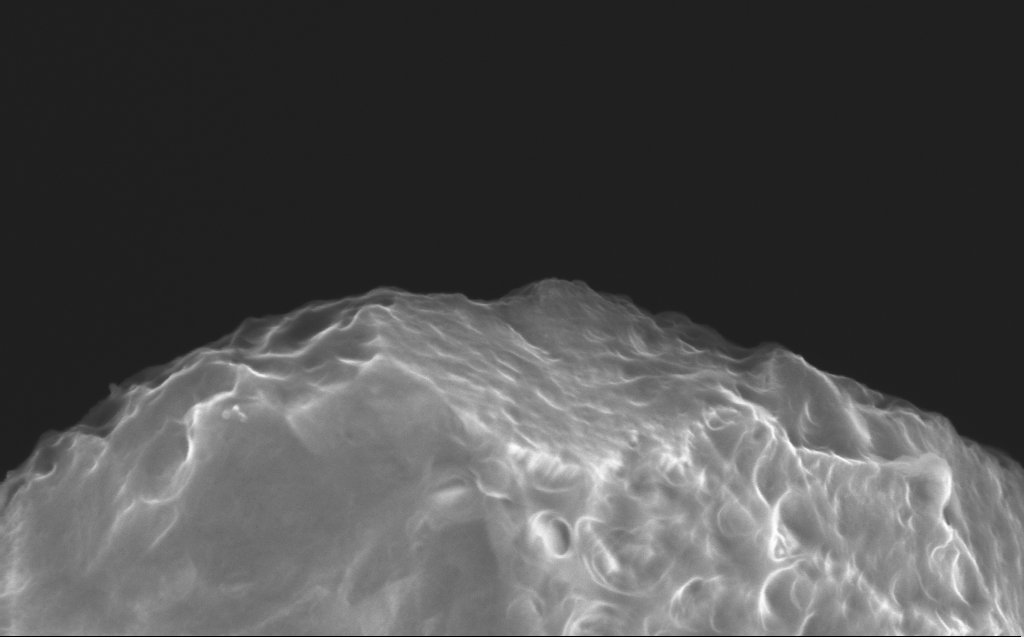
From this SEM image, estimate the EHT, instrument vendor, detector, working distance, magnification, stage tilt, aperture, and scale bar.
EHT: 10 kV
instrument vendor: Zeiss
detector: InLens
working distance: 4 mm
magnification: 137.89 K X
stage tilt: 40°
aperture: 30 µm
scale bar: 200 nm